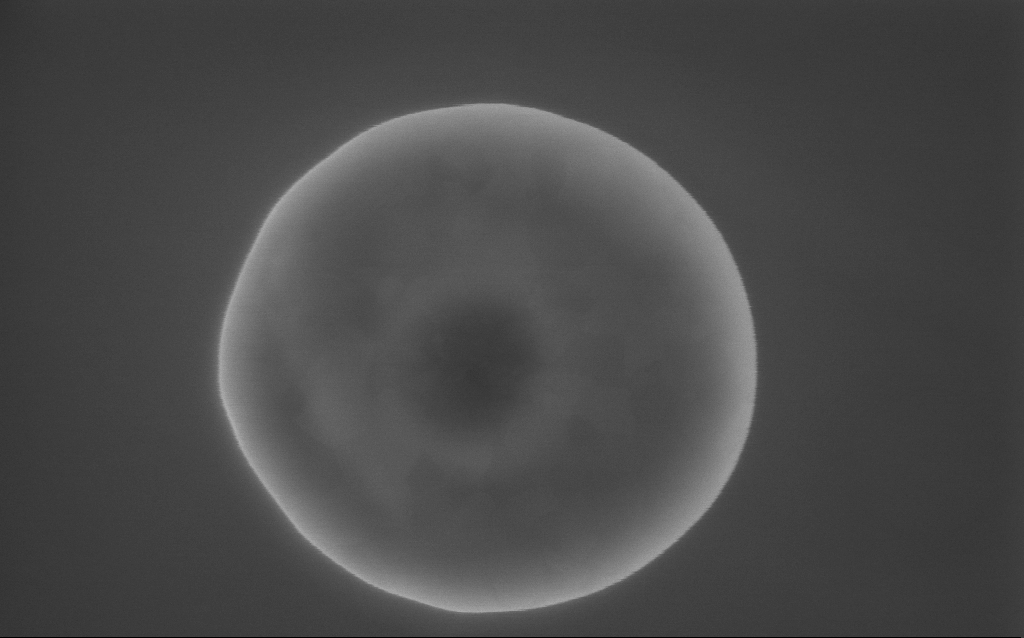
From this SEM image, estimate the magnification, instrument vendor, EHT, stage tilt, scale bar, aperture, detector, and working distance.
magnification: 157 K X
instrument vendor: Zeiss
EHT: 10 kV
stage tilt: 0°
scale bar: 100 nm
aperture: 30 µm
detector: InLens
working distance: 3 mm